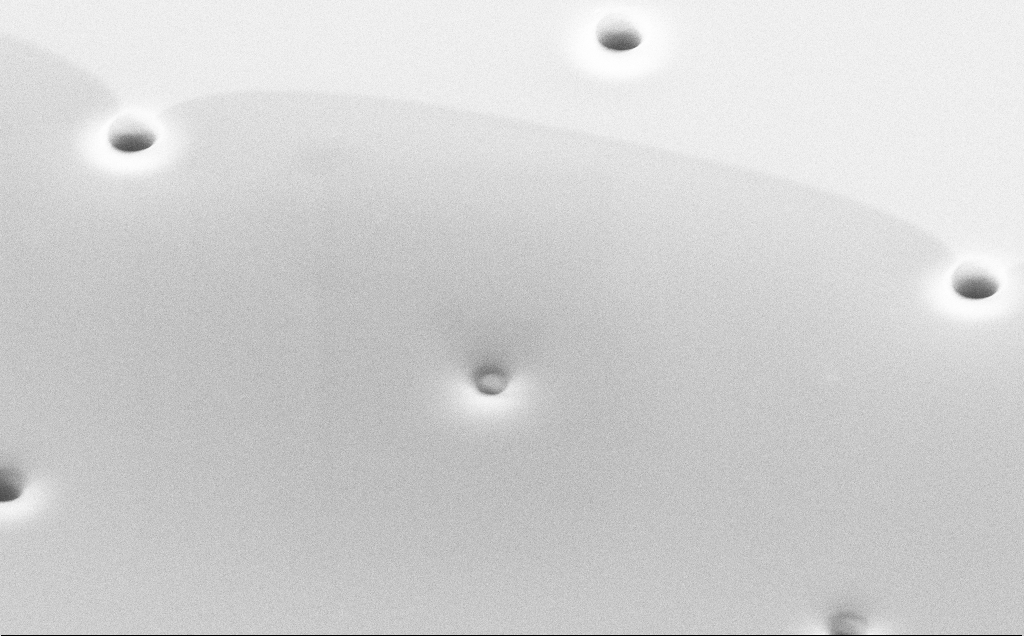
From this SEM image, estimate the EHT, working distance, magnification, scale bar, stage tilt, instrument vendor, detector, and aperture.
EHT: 10 kV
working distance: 13 mm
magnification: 9.16 K X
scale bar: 2000 nm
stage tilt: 45°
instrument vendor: Zeiss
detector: SE2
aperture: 30 µm